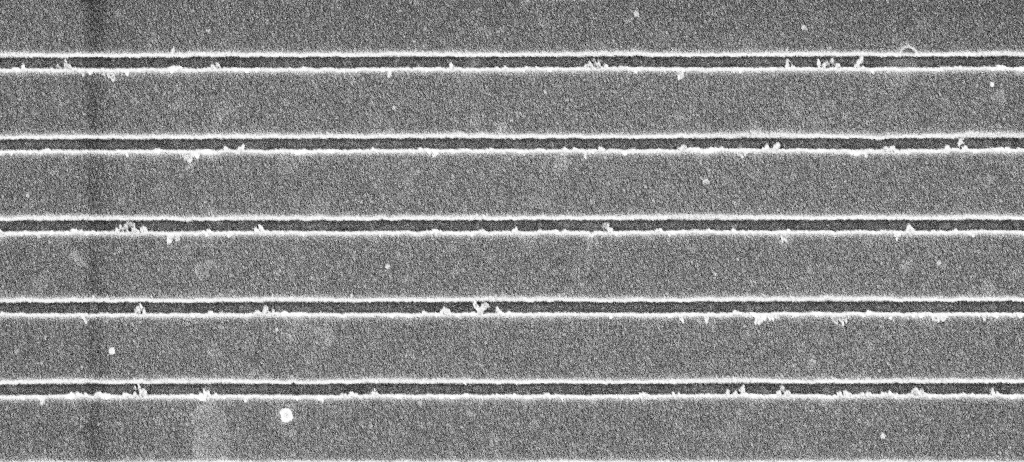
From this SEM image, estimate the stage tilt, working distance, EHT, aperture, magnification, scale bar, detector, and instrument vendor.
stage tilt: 0°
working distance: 5.3 mm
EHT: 5 kV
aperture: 30 µm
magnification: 43.09 K X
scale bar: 1000 nm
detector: InLens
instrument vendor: Zeiss